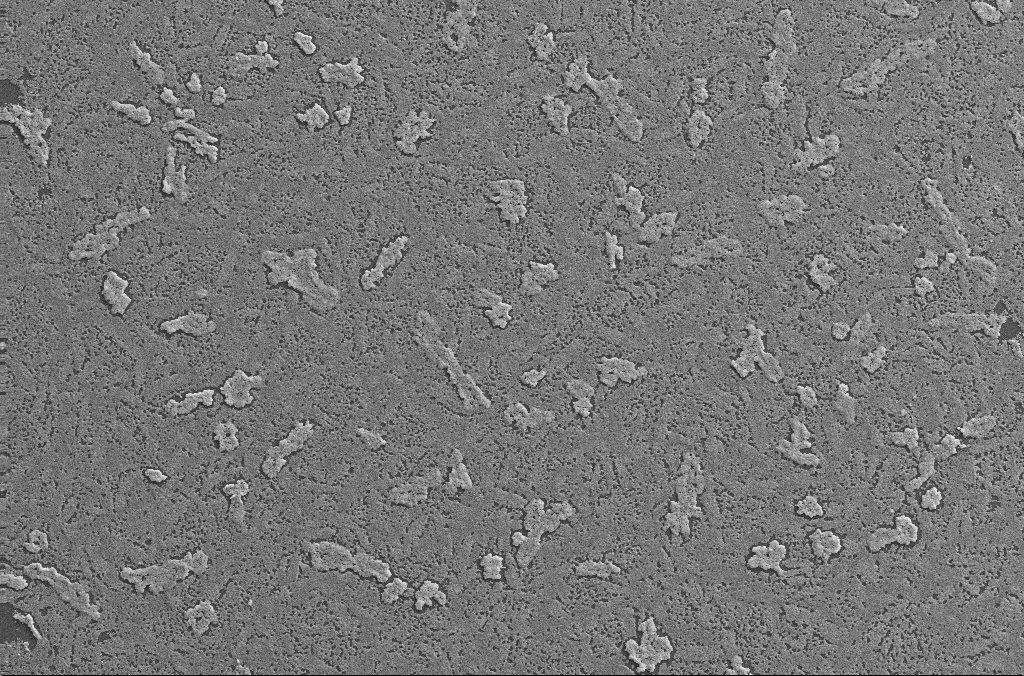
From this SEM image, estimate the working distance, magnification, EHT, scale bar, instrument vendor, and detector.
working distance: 2.8 mm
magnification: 0.5 K X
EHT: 20 kV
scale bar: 100000 nm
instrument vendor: Zeiss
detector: SE2